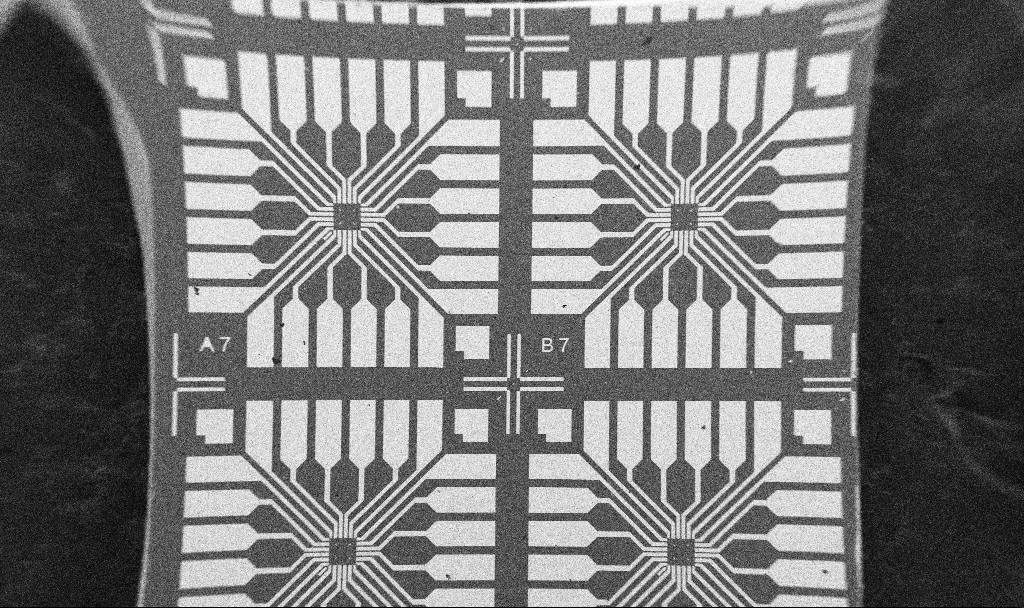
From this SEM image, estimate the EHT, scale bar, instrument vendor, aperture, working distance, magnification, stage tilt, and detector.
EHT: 5 kV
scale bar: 1e+06 nm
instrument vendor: Zeiss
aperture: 30 µm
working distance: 10.7 mm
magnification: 0.061 K X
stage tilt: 0°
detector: SE2